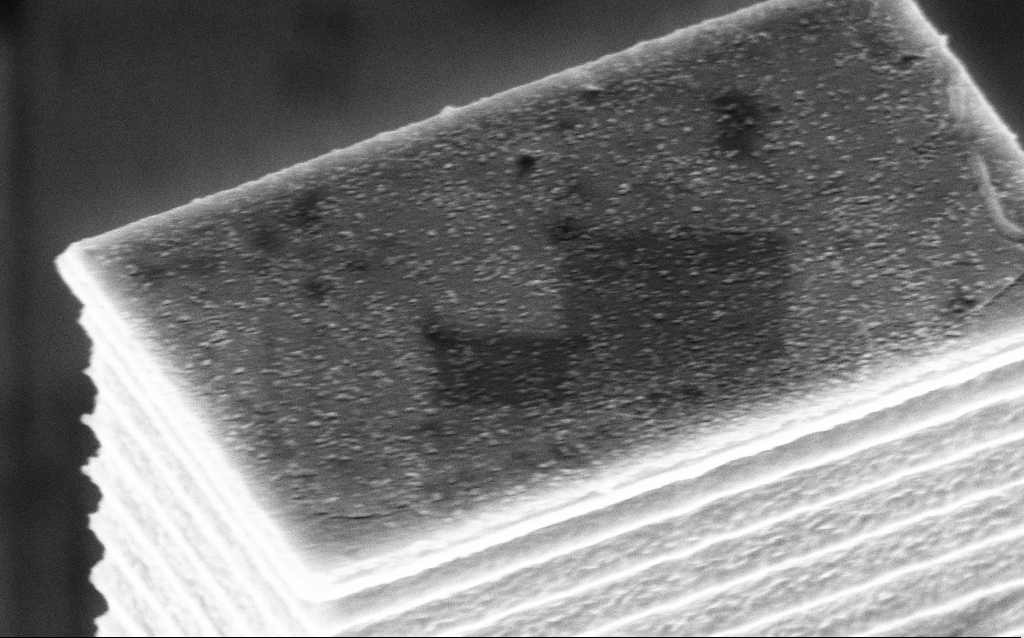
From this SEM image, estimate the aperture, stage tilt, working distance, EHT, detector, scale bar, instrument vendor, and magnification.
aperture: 30 µm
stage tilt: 45°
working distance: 4 mm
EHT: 5 kV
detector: InLens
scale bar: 1000 nm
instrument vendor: Zeiss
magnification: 70.33 K X